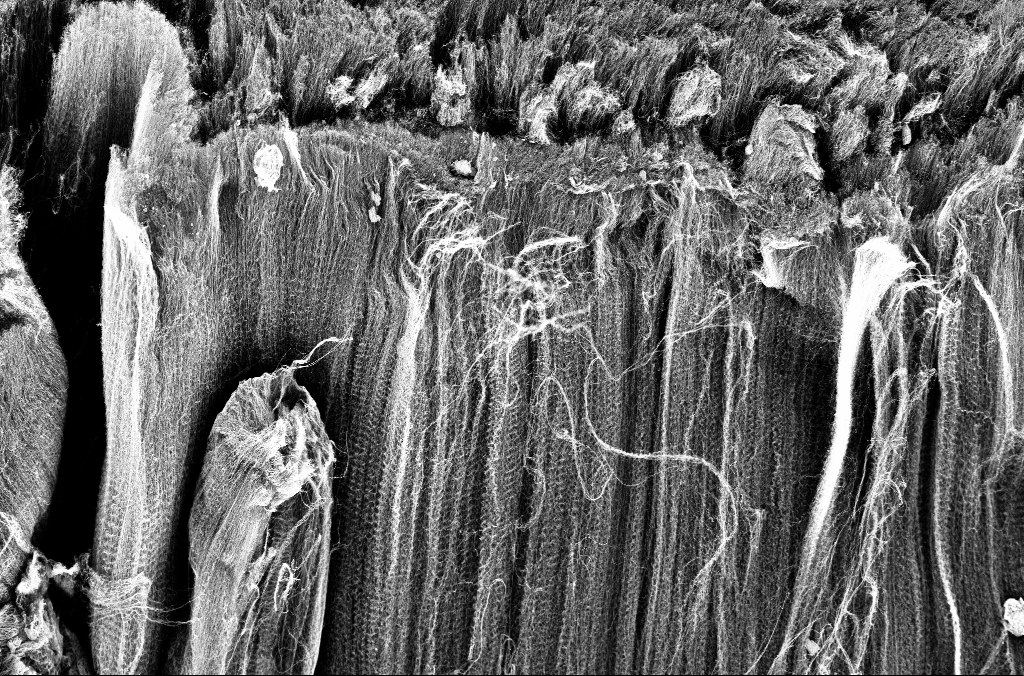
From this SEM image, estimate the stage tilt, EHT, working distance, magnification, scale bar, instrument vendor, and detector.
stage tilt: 45°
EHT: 3 kV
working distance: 3.3 mm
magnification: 2.5 K X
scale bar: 10000 nm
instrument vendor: Zeiss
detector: InLens